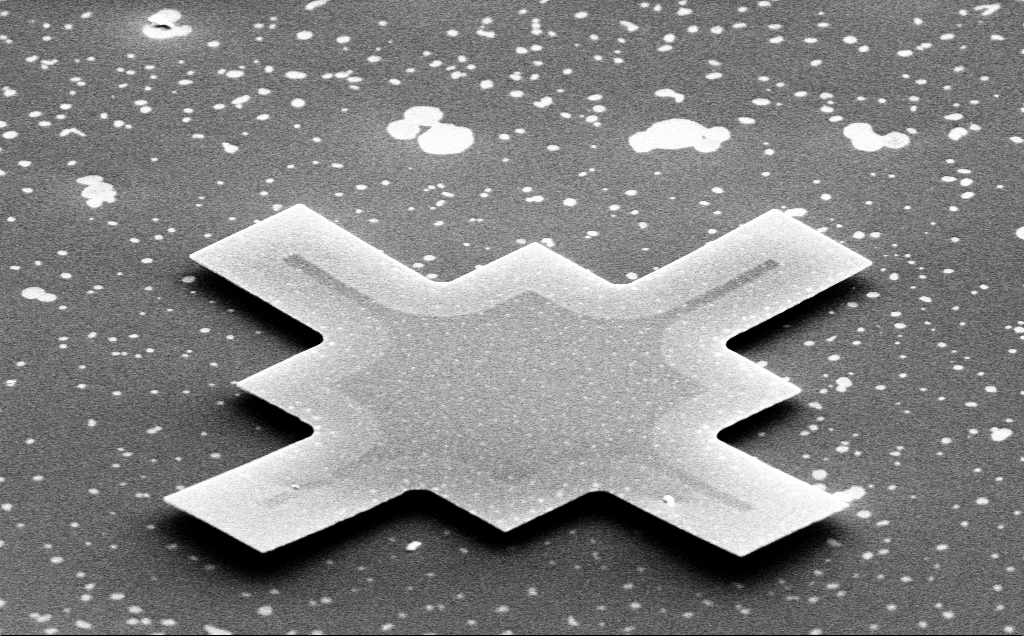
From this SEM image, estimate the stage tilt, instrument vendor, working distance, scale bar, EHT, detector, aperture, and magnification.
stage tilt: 59.7°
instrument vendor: Zeiss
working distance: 14 mm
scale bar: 20000 nm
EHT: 10 kV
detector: SE2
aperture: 30 µm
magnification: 1.55 K X